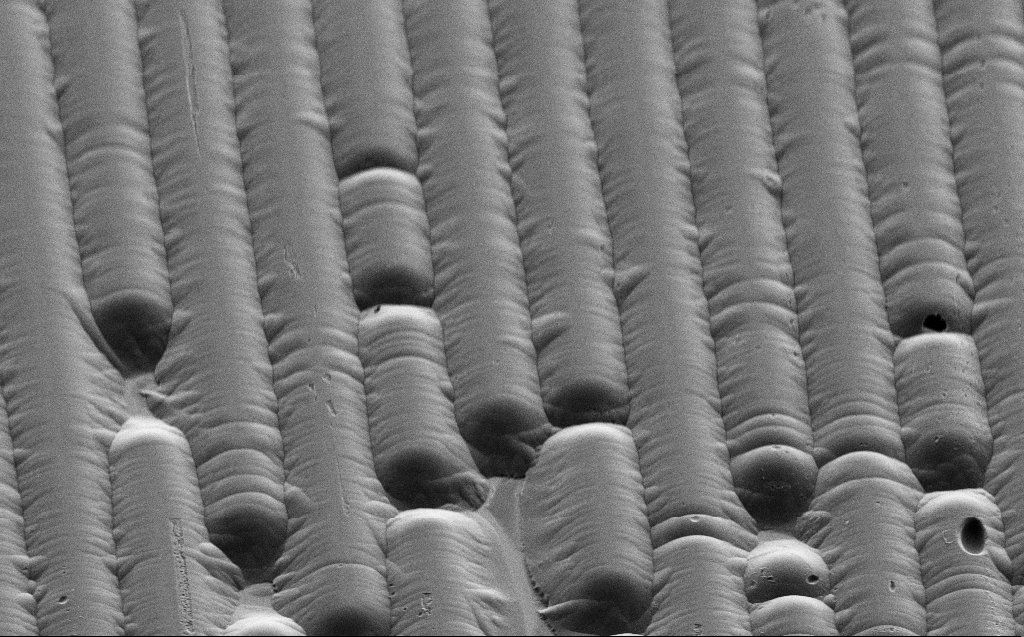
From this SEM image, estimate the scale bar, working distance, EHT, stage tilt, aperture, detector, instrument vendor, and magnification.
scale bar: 10000 nm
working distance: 6 mm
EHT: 3 kV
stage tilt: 45°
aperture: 30 µm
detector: SE2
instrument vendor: Zeiss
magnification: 3.27 K X